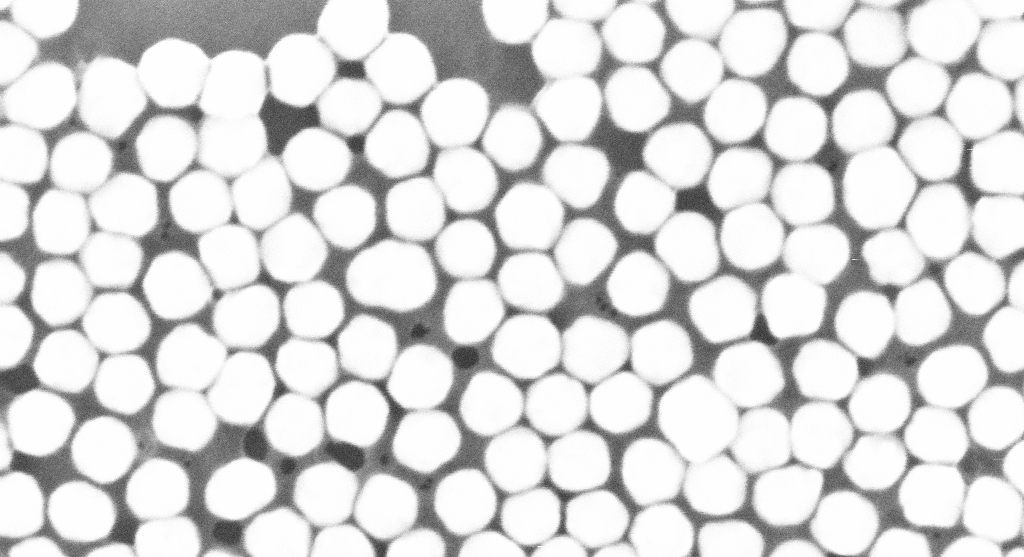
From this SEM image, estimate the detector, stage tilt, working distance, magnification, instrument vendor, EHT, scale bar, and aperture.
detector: InLens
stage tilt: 0°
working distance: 3.1 mm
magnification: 391.37 K X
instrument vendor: Zeiss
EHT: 10 kV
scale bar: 100 nm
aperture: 30 µm